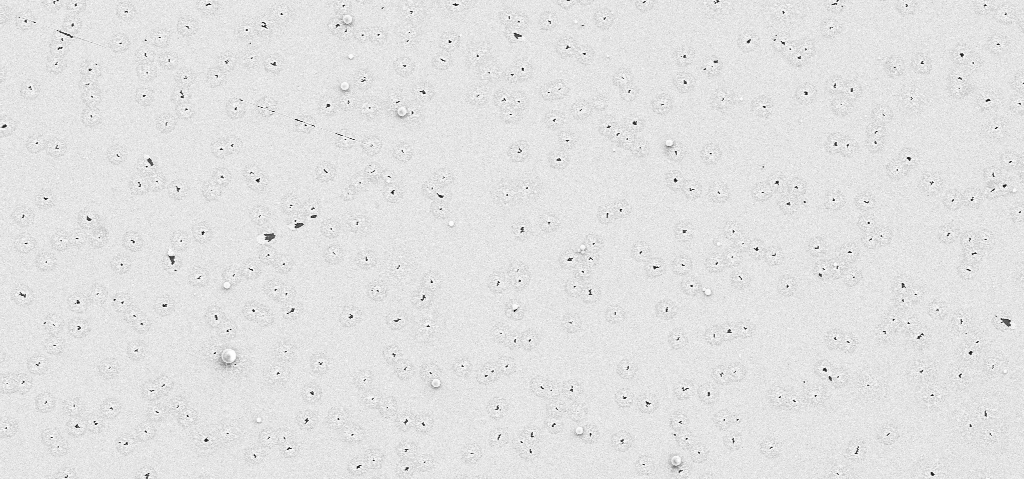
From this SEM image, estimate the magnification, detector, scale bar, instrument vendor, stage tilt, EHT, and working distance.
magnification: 4.2 K X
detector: SE2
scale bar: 10000 nm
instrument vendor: Zeiss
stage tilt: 0°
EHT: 5 kV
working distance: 12 mm